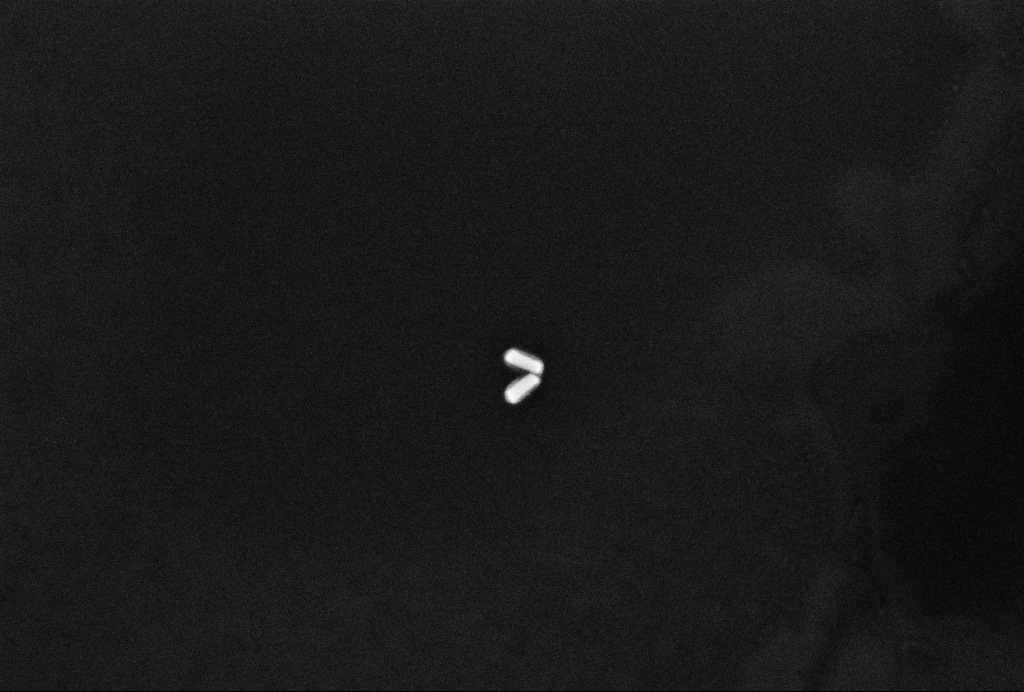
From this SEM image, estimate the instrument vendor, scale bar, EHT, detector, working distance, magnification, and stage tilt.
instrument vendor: Zeiss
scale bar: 100 nm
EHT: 10 kV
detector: InLens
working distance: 3.2 mm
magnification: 200 K X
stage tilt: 0°